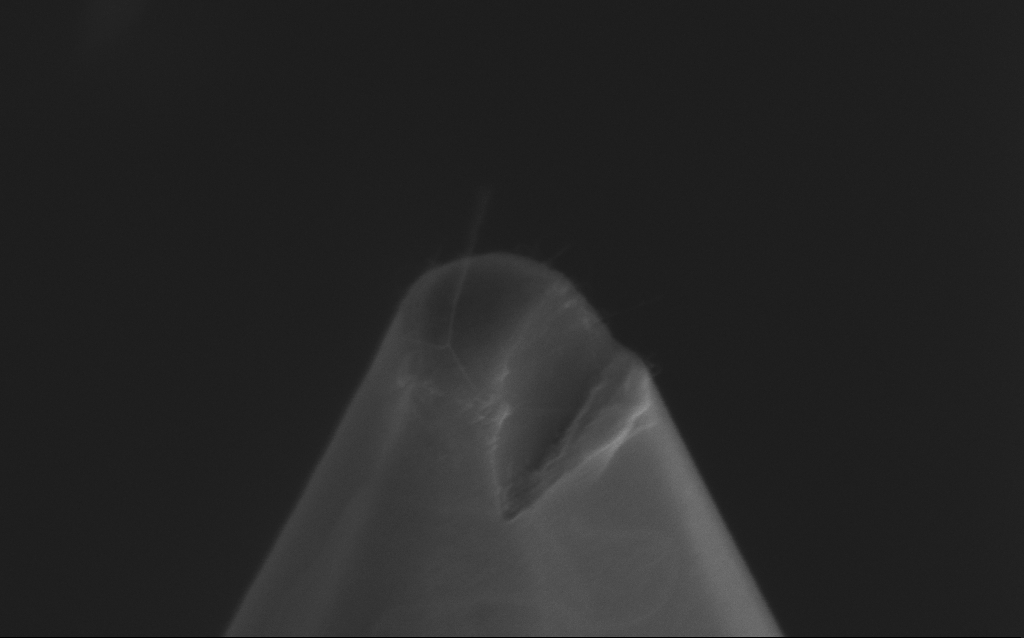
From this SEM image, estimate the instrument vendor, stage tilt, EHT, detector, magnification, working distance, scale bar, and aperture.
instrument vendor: Zeiss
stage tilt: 42.7°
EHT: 5 kV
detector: InLens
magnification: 150.76 K X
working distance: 9 mm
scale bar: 200 nm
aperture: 30 µm